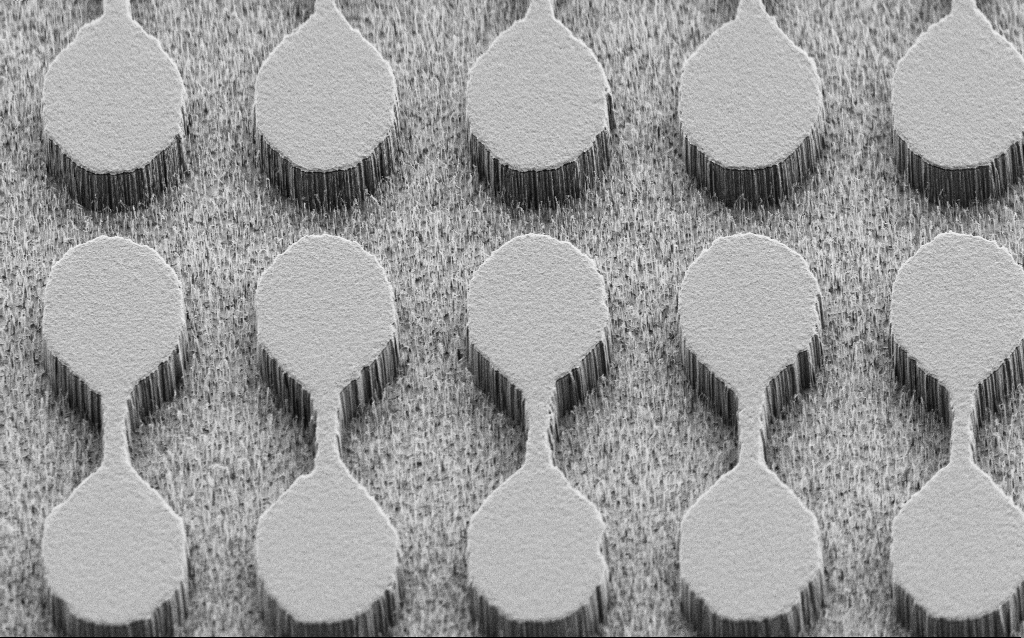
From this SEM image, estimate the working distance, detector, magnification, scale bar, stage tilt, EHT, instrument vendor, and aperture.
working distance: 7 mm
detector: SE2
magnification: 3 K X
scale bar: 20000 nm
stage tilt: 45°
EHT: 2 kV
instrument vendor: Zeiss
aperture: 30 µm